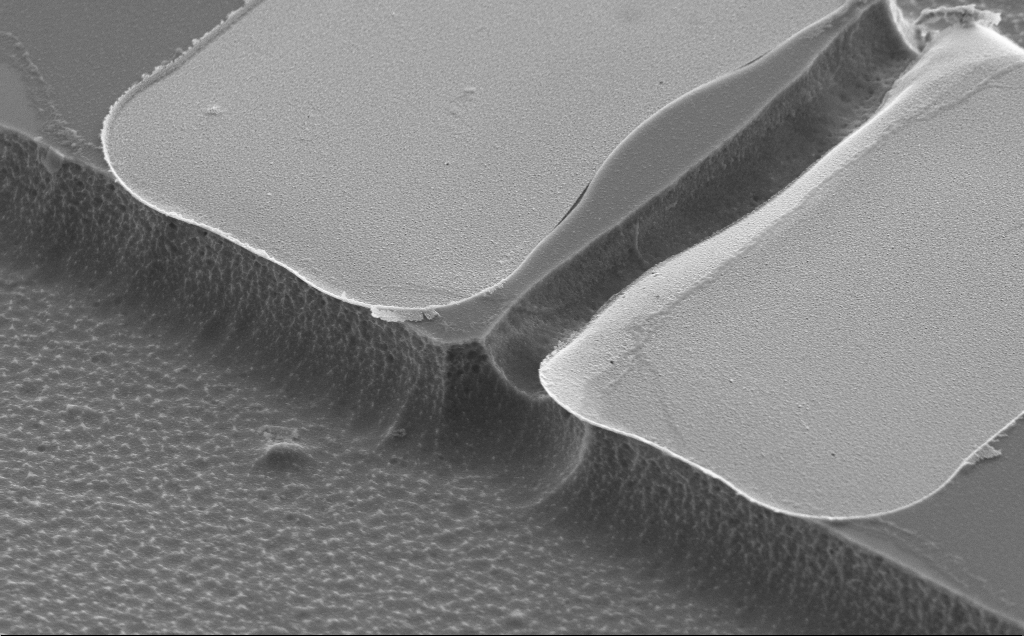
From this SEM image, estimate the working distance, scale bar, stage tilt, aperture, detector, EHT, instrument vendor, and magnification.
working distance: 10 mm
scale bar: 1000 nm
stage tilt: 50°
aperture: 30 µm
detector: SE2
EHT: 5 kV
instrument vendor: Zeiss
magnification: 14.82 K X